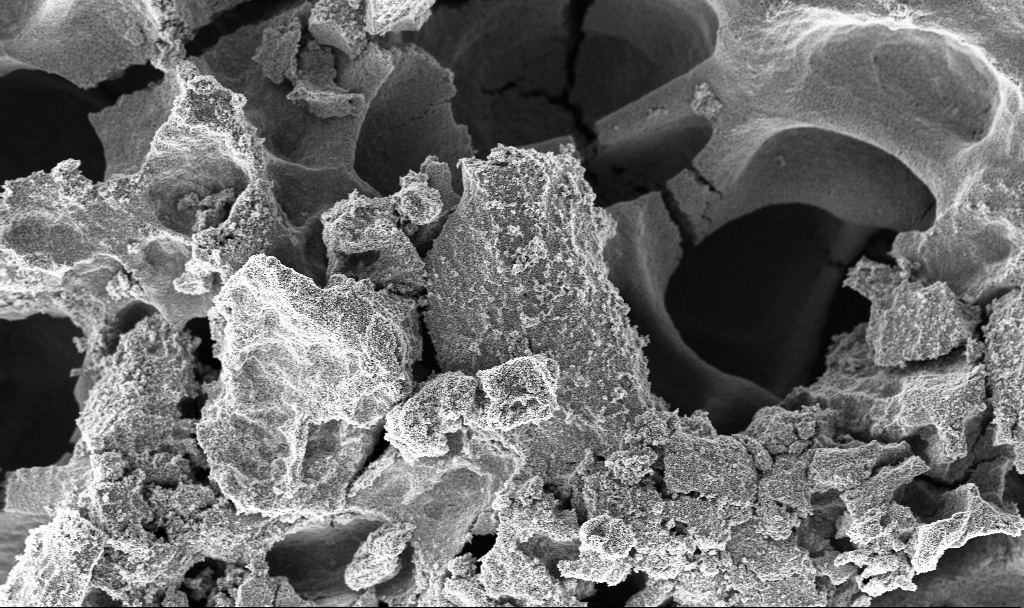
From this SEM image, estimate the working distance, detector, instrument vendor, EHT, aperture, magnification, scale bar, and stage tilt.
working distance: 2.4 mm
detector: InLens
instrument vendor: Zeiss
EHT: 3 kV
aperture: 30 µm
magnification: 3.5 K X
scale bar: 10000 nm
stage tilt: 0°